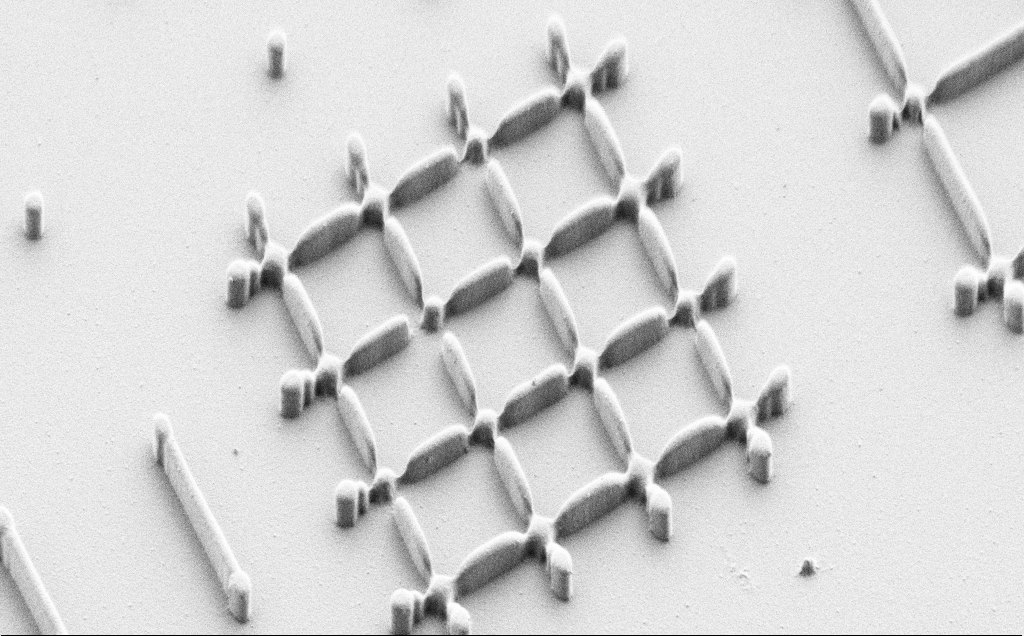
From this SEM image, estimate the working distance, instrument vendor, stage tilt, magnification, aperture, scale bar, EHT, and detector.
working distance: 7 mm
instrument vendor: Zeiss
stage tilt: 45°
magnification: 6.49 K X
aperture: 30 µm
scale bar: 10000 nm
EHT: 1 kV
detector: SE2